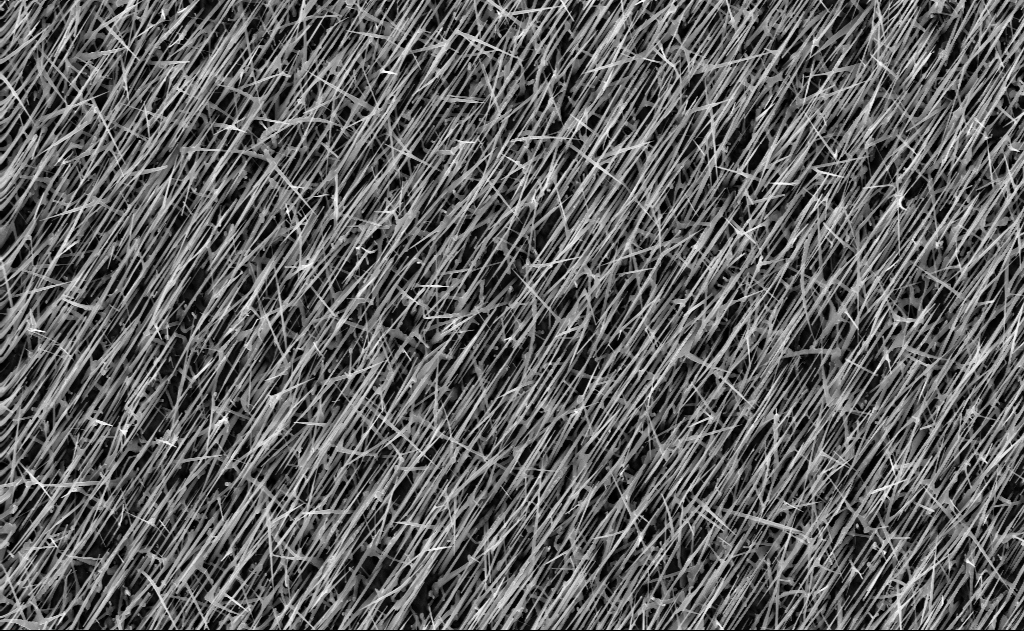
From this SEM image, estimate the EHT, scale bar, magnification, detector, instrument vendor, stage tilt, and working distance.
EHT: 10 kV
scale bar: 2000 nm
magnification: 10 K X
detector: InLens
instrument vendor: Zeiss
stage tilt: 0°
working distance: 7 mm